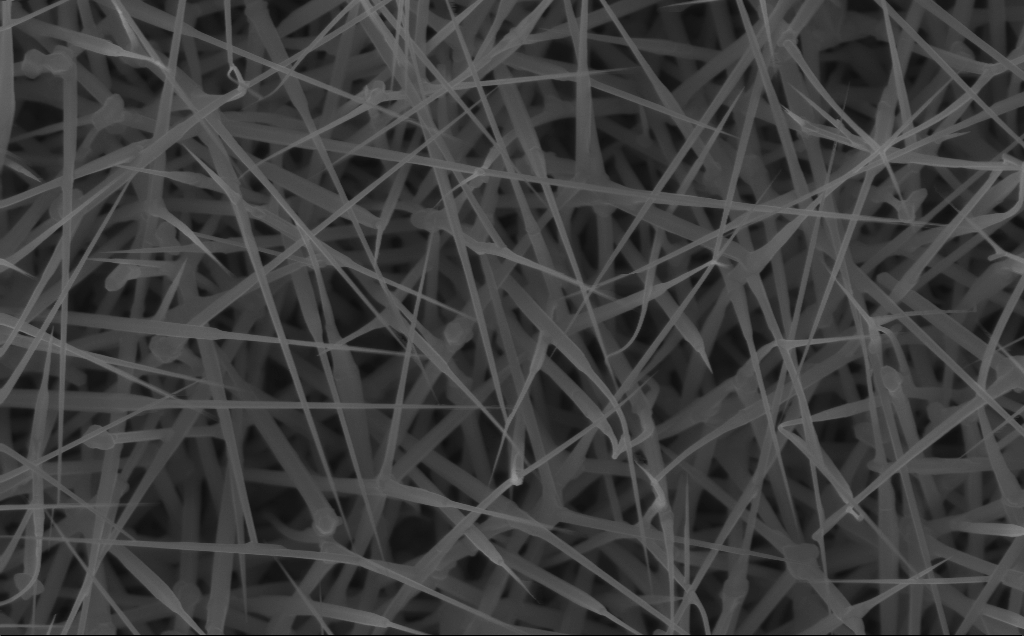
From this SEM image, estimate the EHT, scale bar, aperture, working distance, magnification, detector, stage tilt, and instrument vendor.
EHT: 10 kV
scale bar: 1000 nm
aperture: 30 µm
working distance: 6 mm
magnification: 40 K X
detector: InLens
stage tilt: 0°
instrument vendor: Zeiss